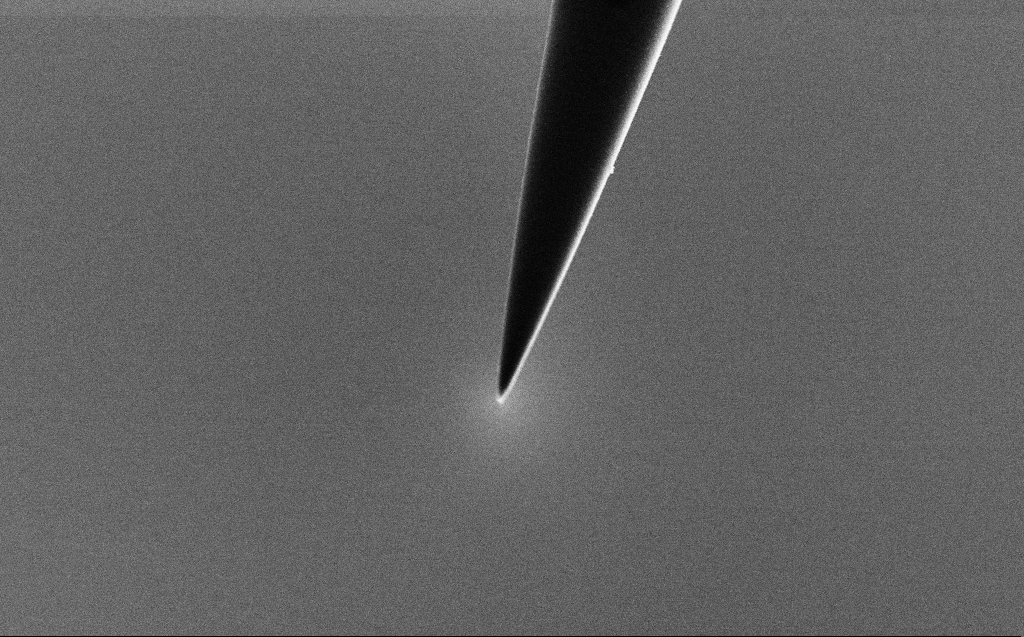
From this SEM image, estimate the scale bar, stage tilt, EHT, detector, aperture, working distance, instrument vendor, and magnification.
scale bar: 1000 nm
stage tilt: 45°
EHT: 3 kV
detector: SE2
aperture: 30 µm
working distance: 3 mm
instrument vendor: Zeiss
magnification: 18.49 K X